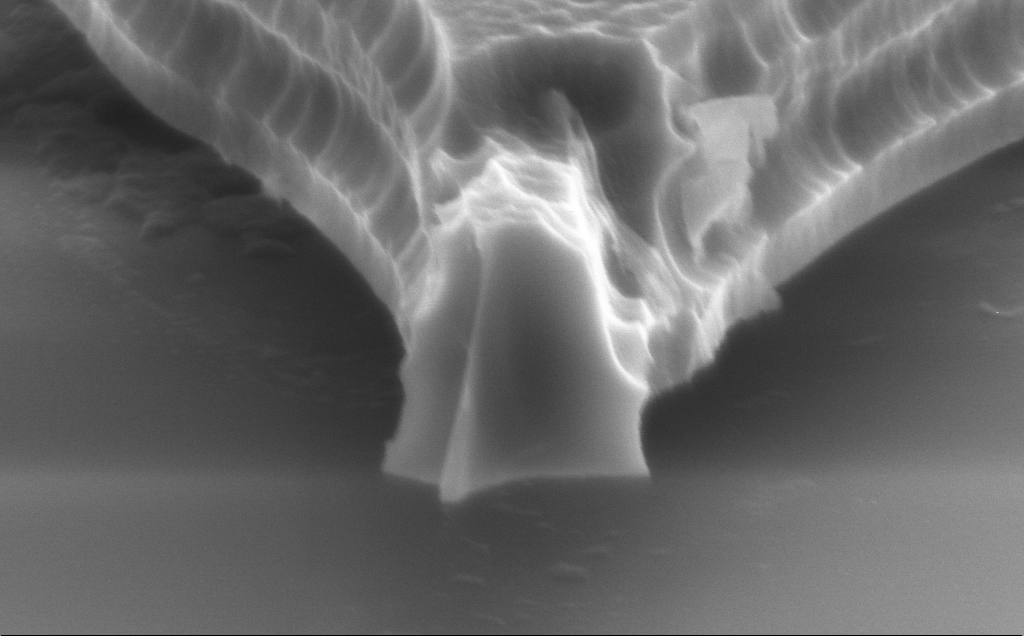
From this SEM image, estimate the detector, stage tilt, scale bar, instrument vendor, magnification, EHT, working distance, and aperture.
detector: SE2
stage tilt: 70°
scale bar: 1000 nm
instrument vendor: Zeiss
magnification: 58.82 K X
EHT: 8 kV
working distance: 12 mm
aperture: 30 µm